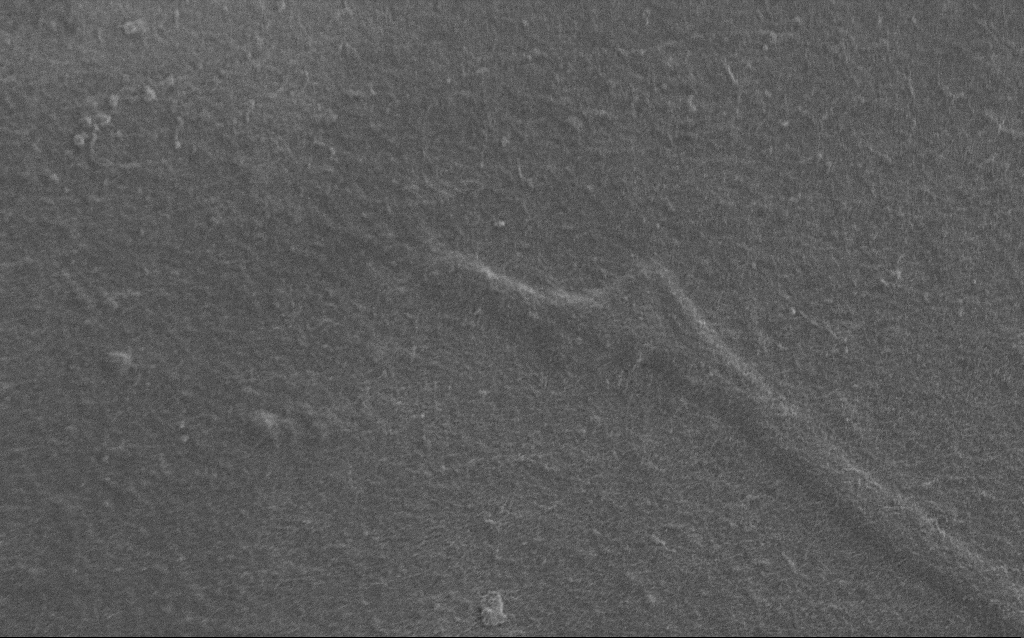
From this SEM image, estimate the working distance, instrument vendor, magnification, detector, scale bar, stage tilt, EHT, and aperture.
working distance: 6 mm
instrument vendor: Zeiss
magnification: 7.5 K X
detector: SE2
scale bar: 2000 nm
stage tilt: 0°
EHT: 0.9 kV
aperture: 30 µm